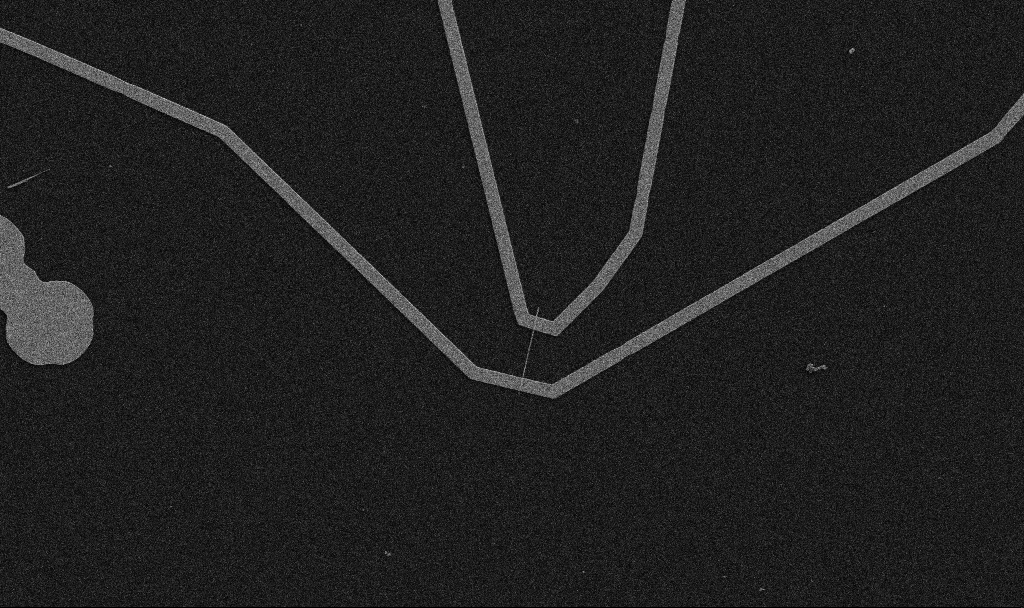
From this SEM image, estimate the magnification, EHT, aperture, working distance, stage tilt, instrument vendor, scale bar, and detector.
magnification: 5 K X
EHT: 5 kV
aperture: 30 µm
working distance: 10.7 mm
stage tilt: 0°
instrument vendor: Zeiss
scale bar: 10000 nm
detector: SE2